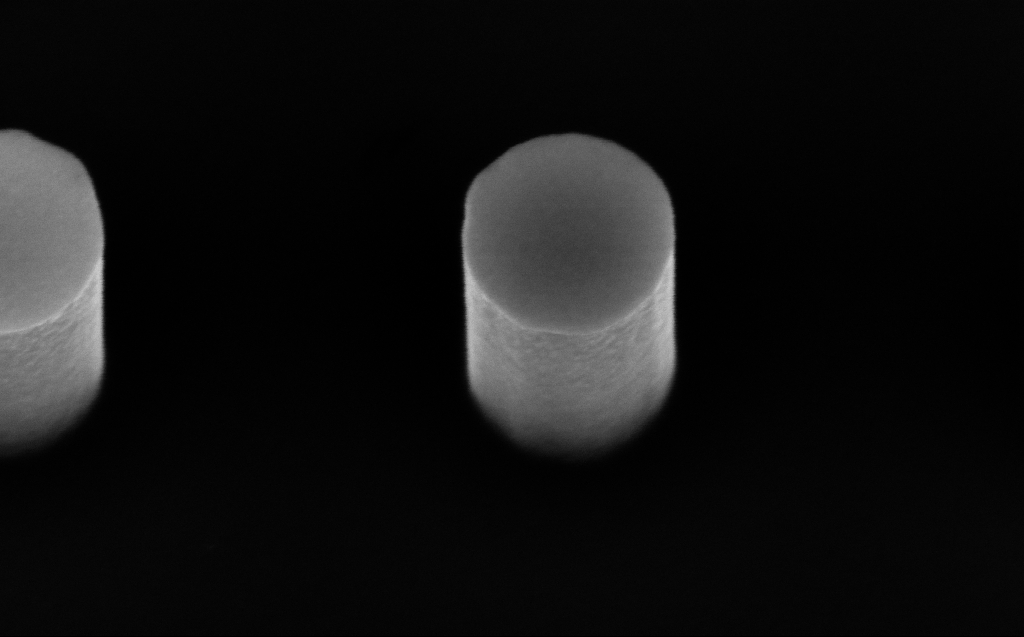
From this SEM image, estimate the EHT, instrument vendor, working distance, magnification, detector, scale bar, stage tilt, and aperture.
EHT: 5 kV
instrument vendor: Zeiss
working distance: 6 mm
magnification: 200 K X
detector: InLens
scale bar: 200 nm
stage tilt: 30°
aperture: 30 µm